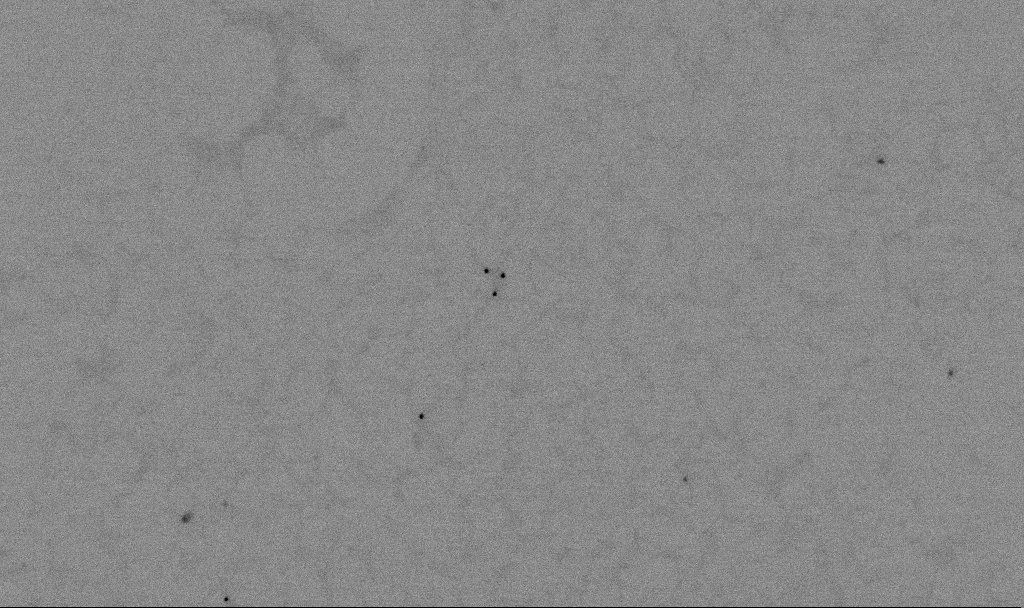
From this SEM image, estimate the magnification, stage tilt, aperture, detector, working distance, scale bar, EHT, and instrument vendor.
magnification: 60 K X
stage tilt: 0°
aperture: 30 µm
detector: SE2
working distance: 5.5 mm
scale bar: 1000 nm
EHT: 2 kV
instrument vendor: Zeiss